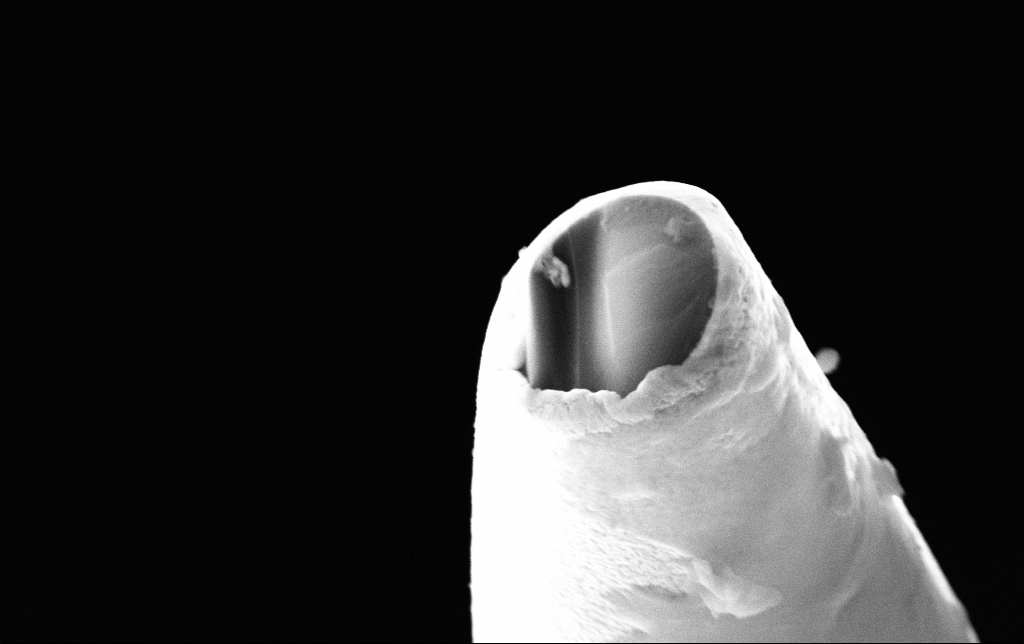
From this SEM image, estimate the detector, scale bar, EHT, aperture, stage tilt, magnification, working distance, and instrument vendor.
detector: SE2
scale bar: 1000 nm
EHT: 10 kV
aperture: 30 µm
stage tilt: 69.3°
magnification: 41.21 K X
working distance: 9.1 mm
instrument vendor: Zeiss